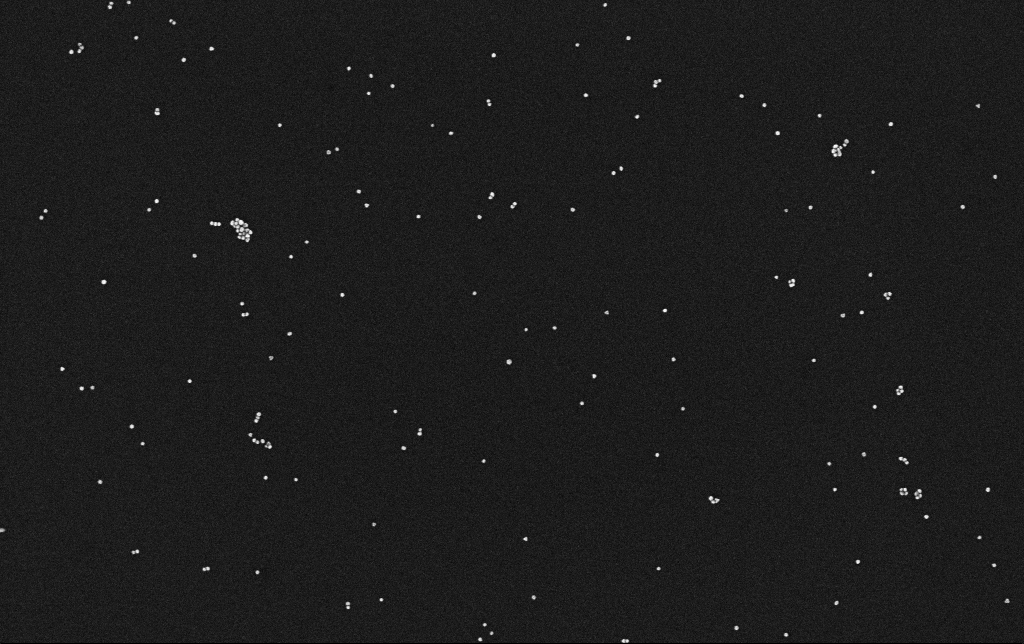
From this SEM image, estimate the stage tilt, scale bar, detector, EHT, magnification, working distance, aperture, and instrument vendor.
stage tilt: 0°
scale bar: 200 nm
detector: InLens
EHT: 10 kV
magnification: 100 K X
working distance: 3.1 mm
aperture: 30 µm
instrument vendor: Zeiss